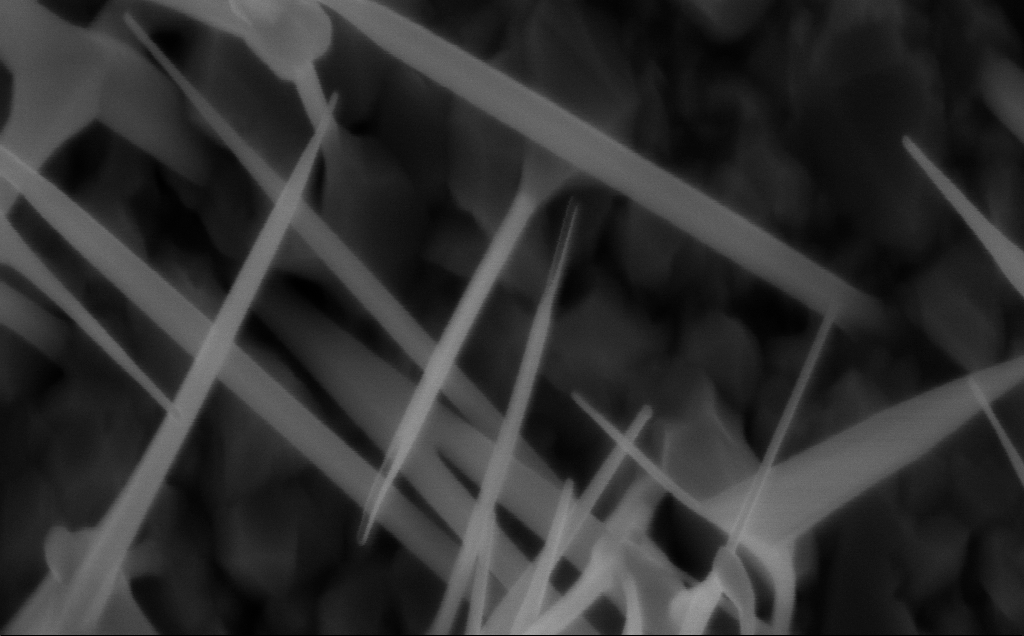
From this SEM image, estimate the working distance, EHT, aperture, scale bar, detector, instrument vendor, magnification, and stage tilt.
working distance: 5 mm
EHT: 5 kV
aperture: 30 µm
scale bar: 100 nm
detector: InLens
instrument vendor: Zeiss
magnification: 150 K X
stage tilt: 0°